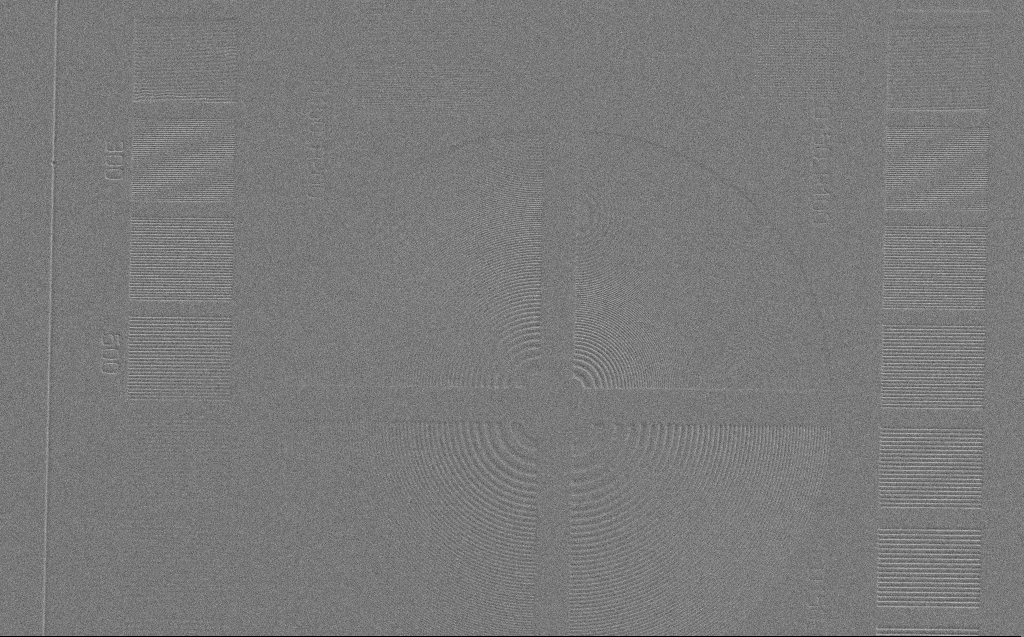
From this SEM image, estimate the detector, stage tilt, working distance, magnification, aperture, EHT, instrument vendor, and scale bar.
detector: SE2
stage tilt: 0°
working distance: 3 mm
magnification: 1.27 K X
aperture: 30 µm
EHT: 3 kV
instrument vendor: Zeiss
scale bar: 20000 nm